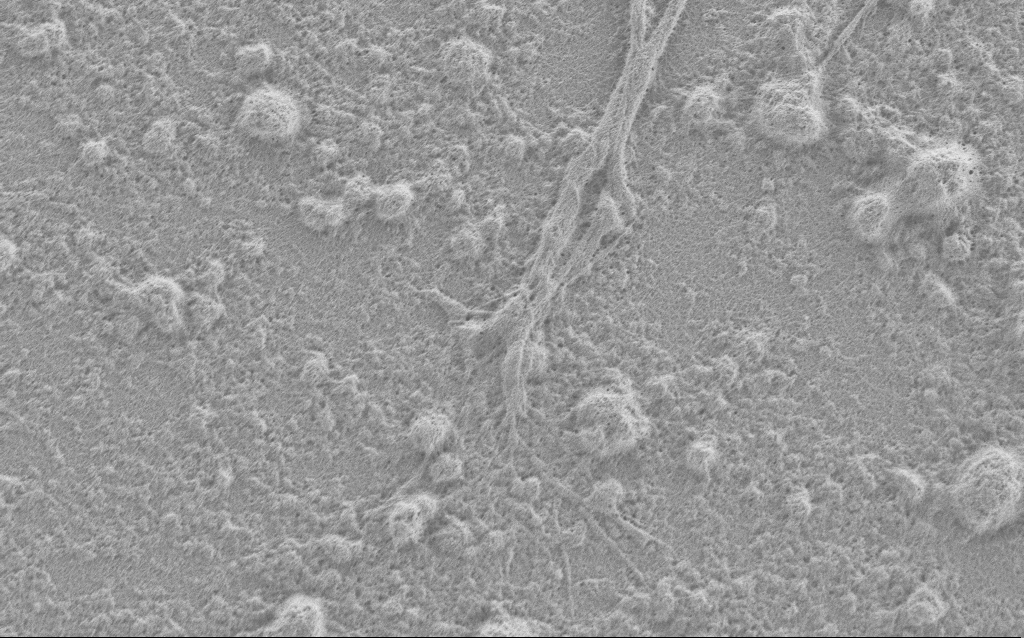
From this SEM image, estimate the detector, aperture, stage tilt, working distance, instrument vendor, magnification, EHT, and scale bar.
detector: SE2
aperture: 30 µm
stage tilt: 0°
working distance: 4 mm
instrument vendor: Zeiss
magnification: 7.5 K X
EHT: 0.9 kV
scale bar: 2000 nm